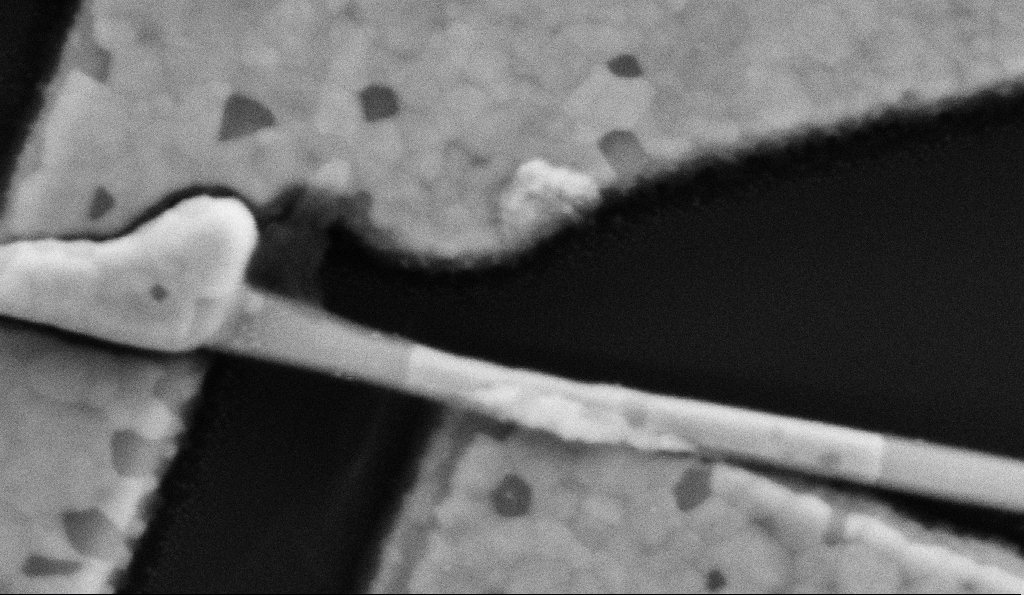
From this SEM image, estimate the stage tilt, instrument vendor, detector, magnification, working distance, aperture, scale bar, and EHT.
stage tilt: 0°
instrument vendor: Zeiss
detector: SE2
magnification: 200 K X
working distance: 8.5 mm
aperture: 30 µm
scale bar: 200 nm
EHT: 5 kV